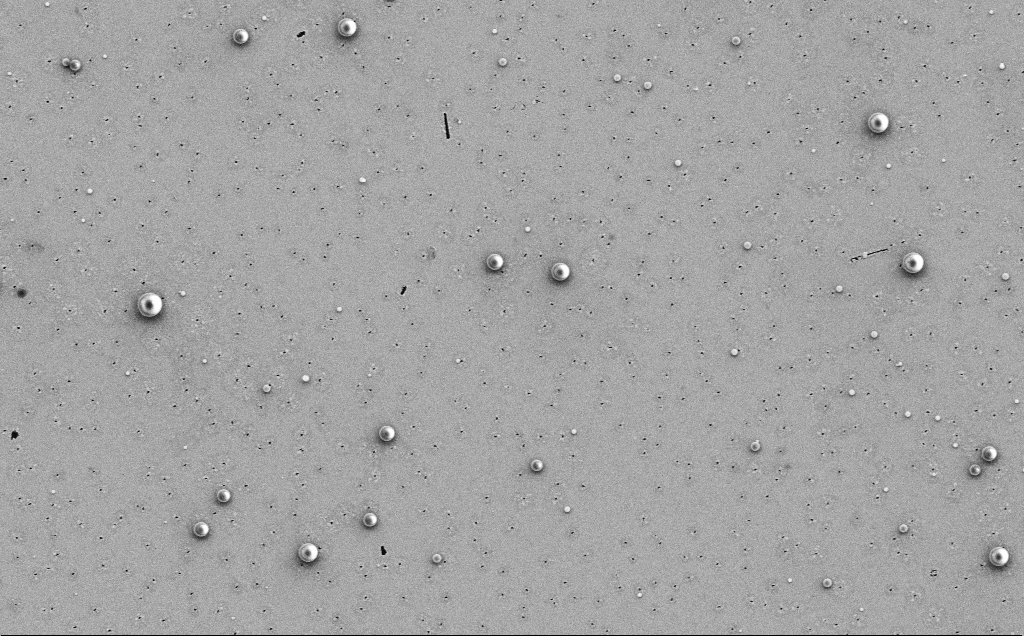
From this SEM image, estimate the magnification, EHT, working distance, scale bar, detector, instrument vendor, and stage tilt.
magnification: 4.2 K X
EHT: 5 kV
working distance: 12 mm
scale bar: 10000 nm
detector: SE2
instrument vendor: Zeiss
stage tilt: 0°